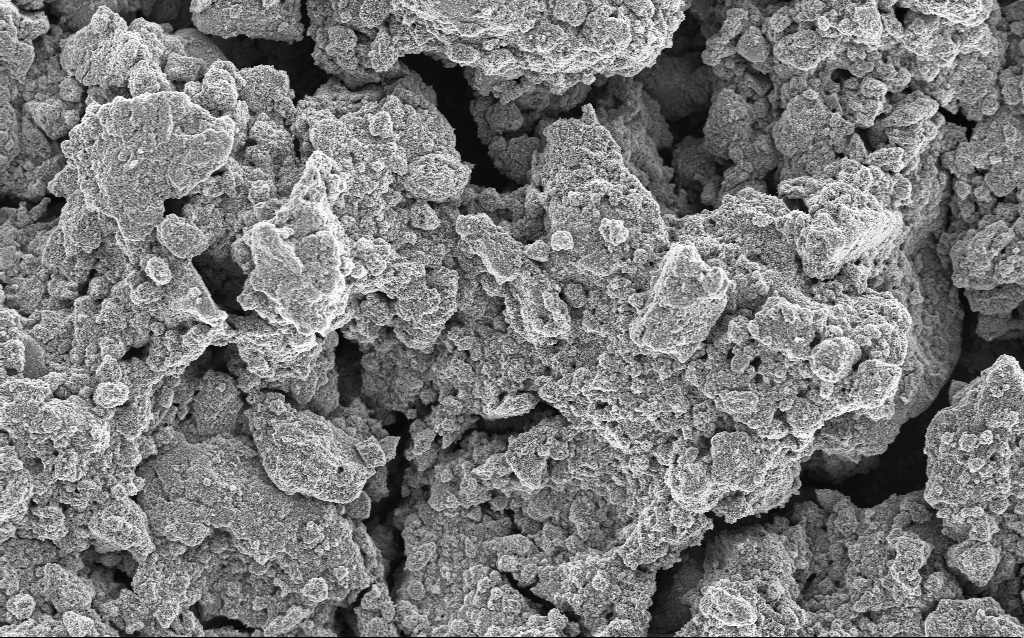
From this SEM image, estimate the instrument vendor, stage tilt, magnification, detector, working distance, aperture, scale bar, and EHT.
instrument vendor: Zeiss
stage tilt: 0°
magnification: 1.23 K X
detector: InLens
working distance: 4.5 mm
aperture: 30 µm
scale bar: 10000 nm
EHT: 5 kV